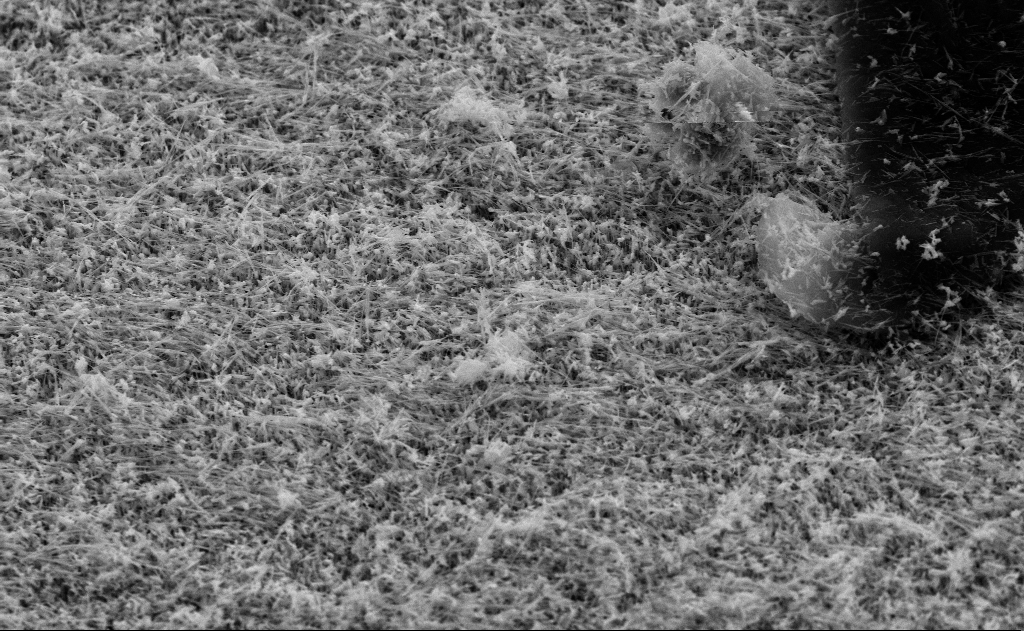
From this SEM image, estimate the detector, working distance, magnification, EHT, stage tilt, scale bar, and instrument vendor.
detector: SE2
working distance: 11 mm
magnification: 10 K X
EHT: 10 kV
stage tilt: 45°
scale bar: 2000 nm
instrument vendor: Zeiss